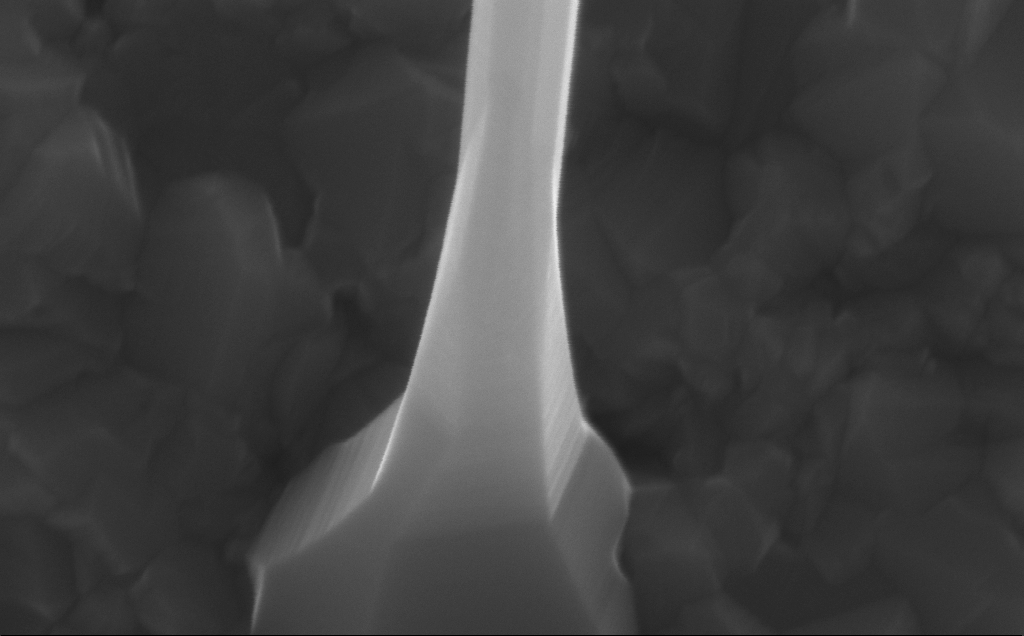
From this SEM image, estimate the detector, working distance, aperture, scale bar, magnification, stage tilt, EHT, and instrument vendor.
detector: InLens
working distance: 5 mm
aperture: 30 µm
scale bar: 200 nm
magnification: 227.85 K X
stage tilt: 0°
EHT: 10 kV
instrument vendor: Zeiss